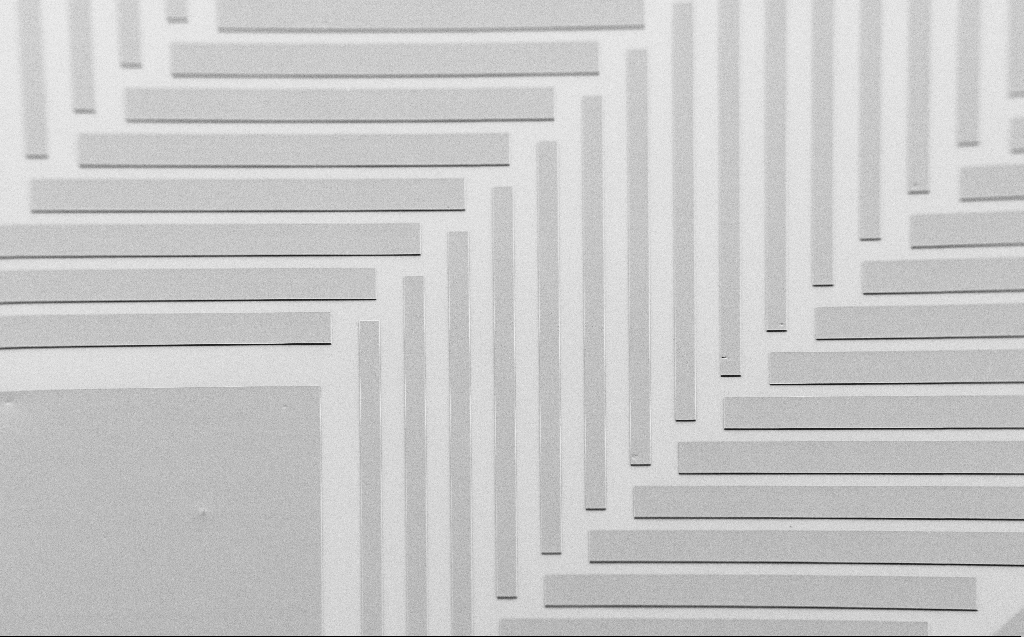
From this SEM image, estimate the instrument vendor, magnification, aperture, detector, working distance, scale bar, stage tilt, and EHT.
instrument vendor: Zeiss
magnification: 0.223 K X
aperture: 30 µm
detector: SE2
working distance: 6 mm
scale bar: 200000 nm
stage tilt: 45°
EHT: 1.7 kV